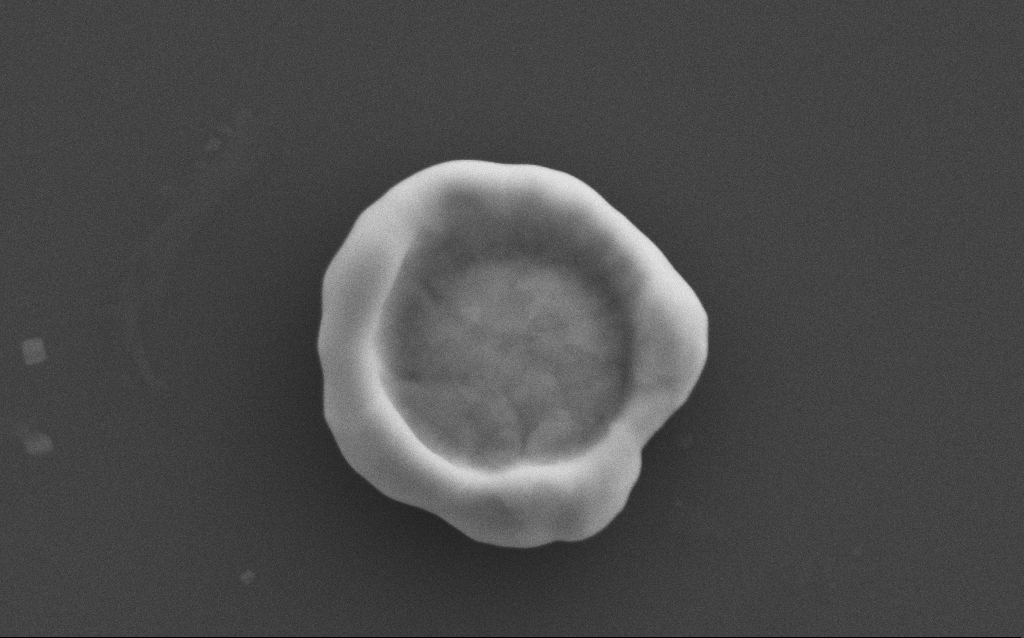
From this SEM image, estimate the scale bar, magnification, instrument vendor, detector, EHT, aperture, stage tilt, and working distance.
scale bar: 1000 nm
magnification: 51.5 K X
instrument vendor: Zeiss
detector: SE2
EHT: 10 kV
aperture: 30 µm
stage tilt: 0°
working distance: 4 mm